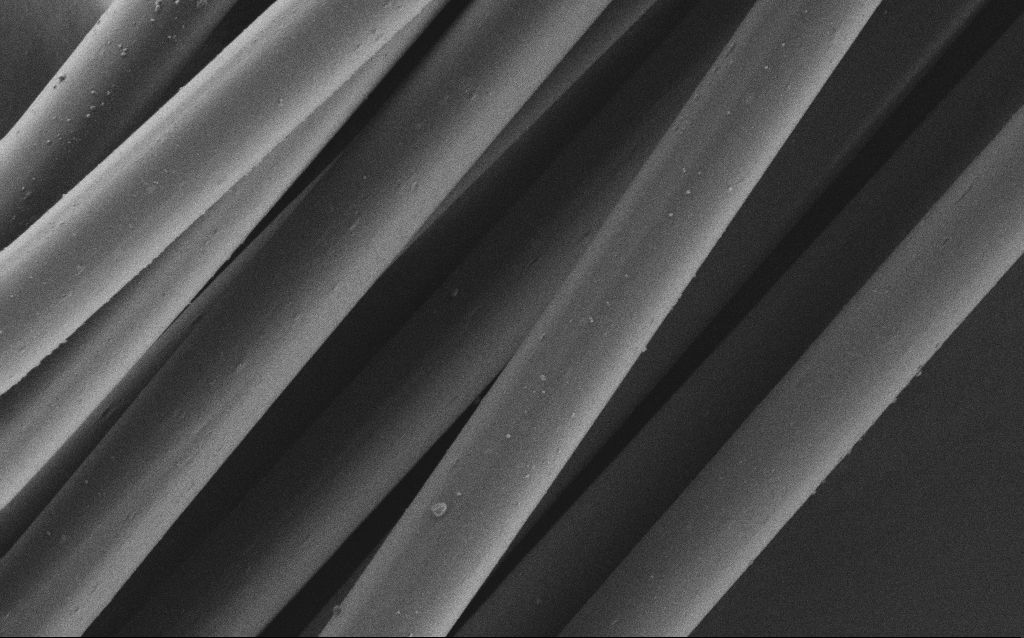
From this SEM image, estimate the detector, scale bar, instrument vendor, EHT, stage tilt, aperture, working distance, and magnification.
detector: SE2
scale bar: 20000 nm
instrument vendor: Zeiss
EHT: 1 kV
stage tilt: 0°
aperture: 30 µm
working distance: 5 mm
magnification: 2.88 K X